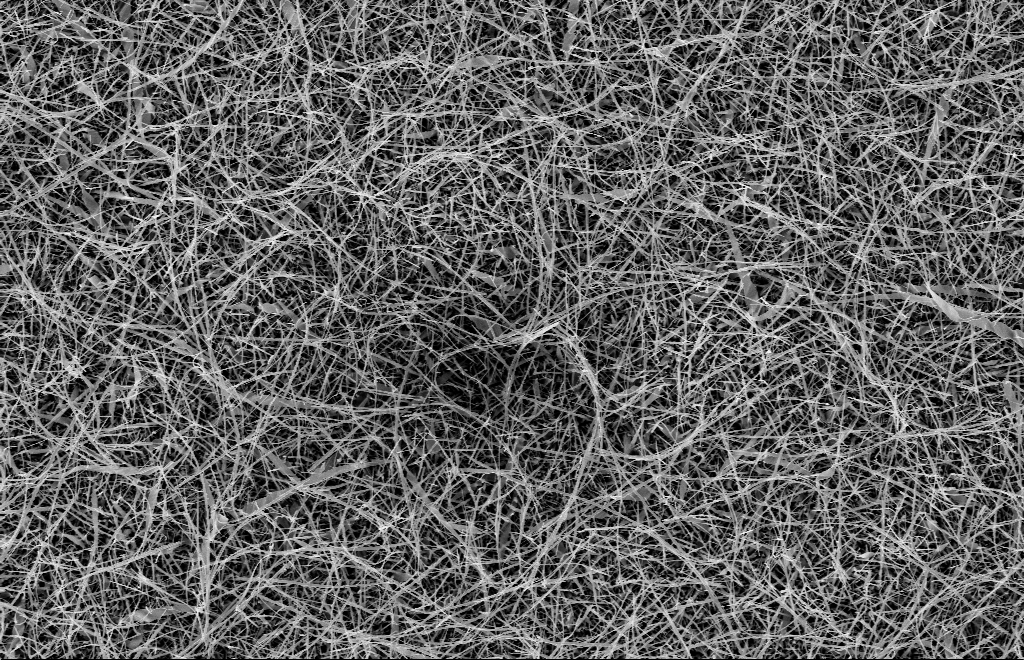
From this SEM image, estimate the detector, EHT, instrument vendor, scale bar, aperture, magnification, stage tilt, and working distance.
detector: InLens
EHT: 10 kV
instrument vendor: Zeiss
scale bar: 2000 nm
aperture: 30 µm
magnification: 5 K X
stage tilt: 0°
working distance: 14 mm